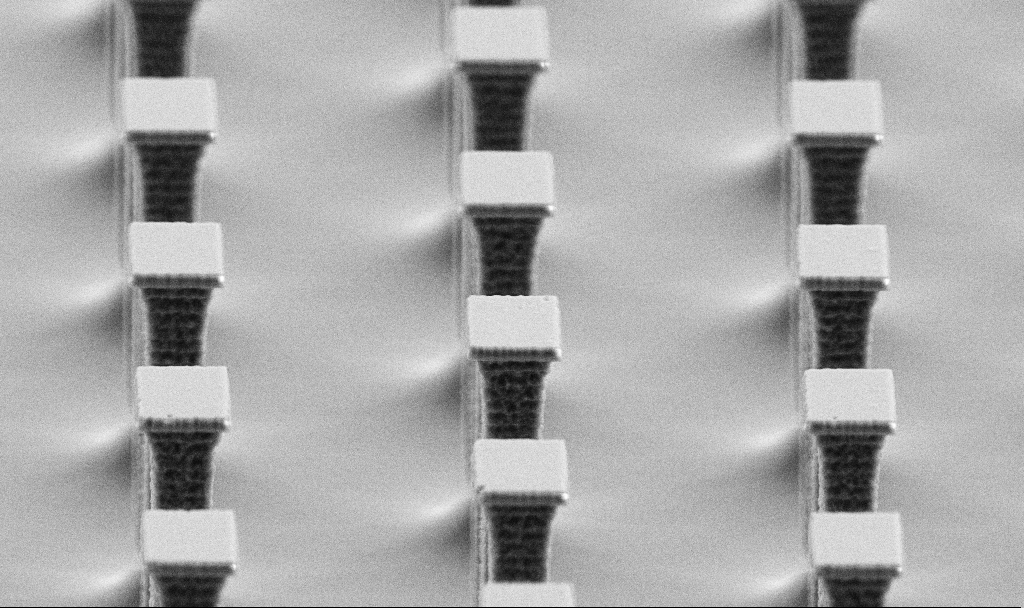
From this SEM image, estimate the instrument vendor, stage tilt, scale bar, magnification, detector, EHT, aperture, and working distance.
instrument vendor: Zeiss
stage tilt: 70°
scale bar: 2000 nm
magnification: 11.74 K X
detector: SE2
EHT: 5 kV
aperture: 30 µm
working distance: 6 mm